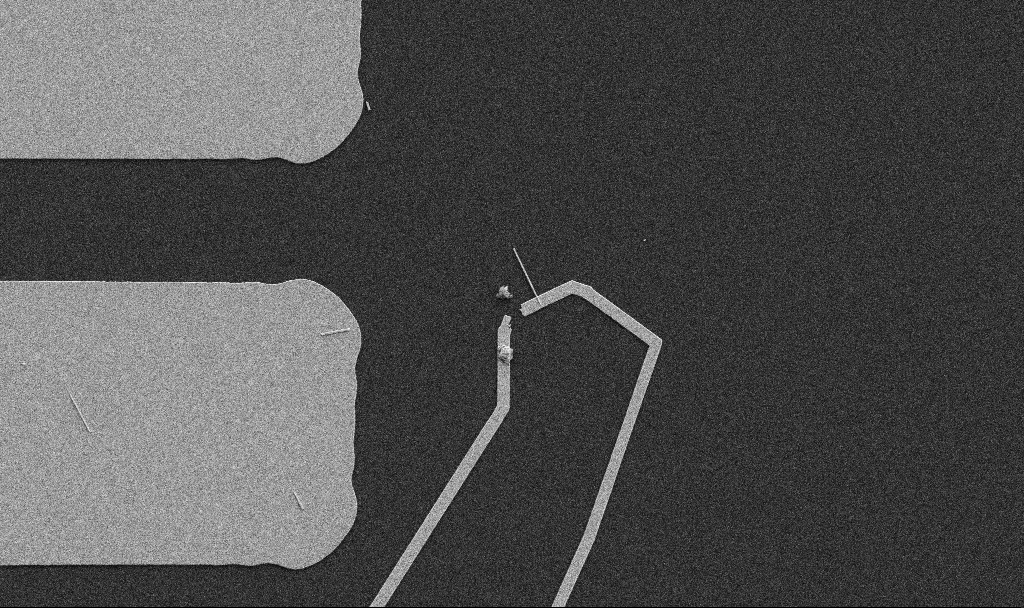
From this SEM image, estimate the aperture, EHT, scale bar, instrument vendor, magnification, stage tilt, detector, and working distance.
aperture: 30 µm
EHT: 5 kV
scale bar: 10000 nm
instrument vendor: Zeiss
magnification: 5 K X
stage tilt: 0°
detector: SE2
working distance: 10.7 mm